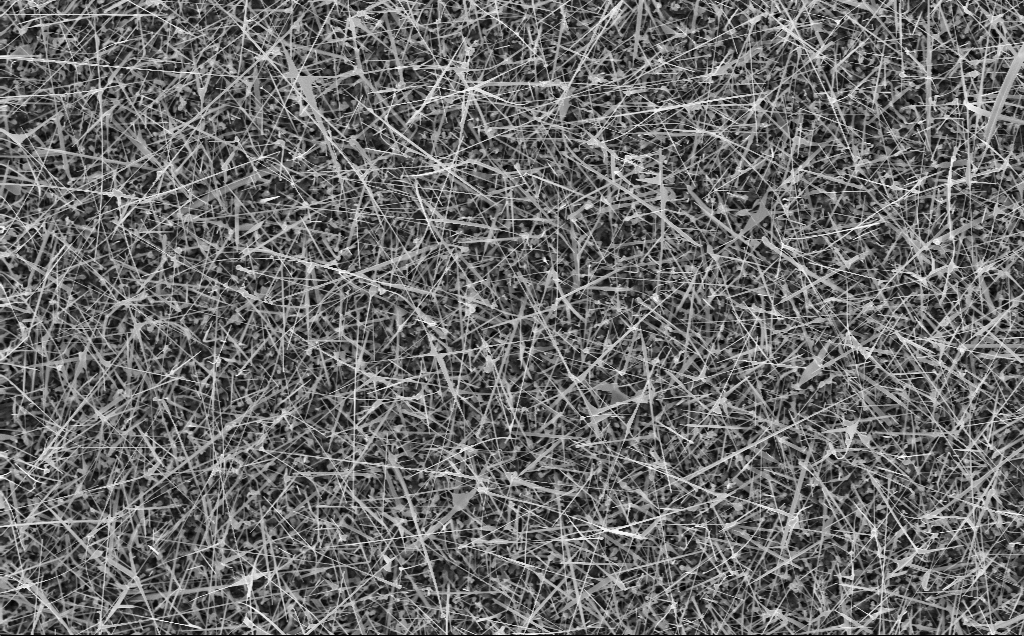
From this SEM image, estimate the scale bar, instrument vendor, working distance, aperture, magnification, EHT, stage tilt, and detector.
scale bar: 2000 nm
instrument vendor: Zeiss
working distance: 8 mm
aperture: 30 µm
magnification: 10 K X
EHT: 10 kV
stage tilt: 0°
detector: InLens